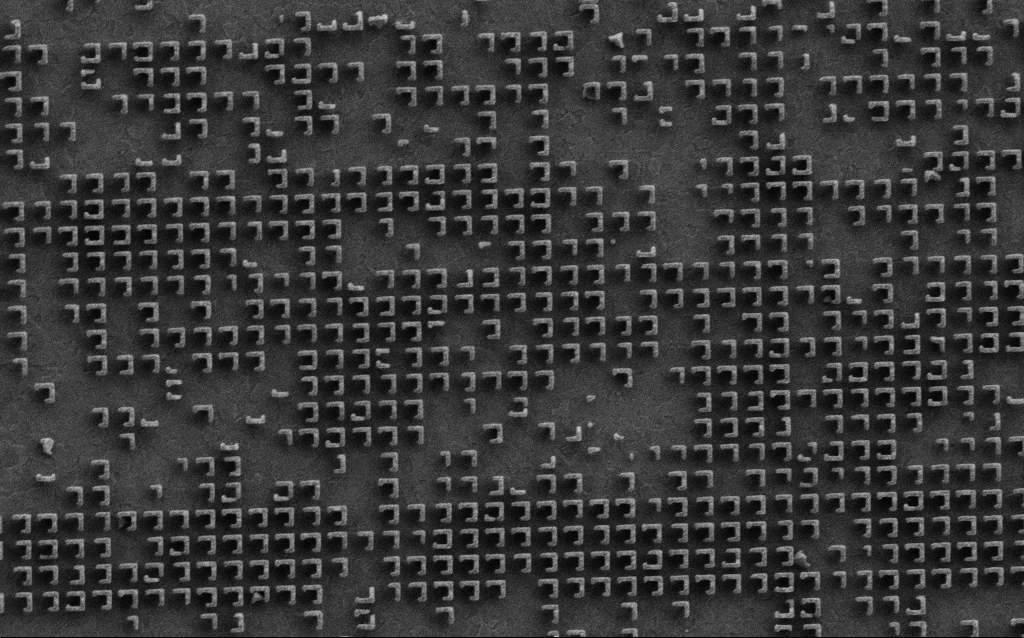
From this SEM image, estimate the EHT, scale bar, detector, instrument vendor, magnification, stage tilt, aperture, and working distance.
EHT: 3 kV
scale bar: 2000 nm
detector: SE2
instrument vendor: Zeiss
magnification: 20.88 K X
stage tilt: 0°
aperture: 30 µm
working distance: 6.6 mm